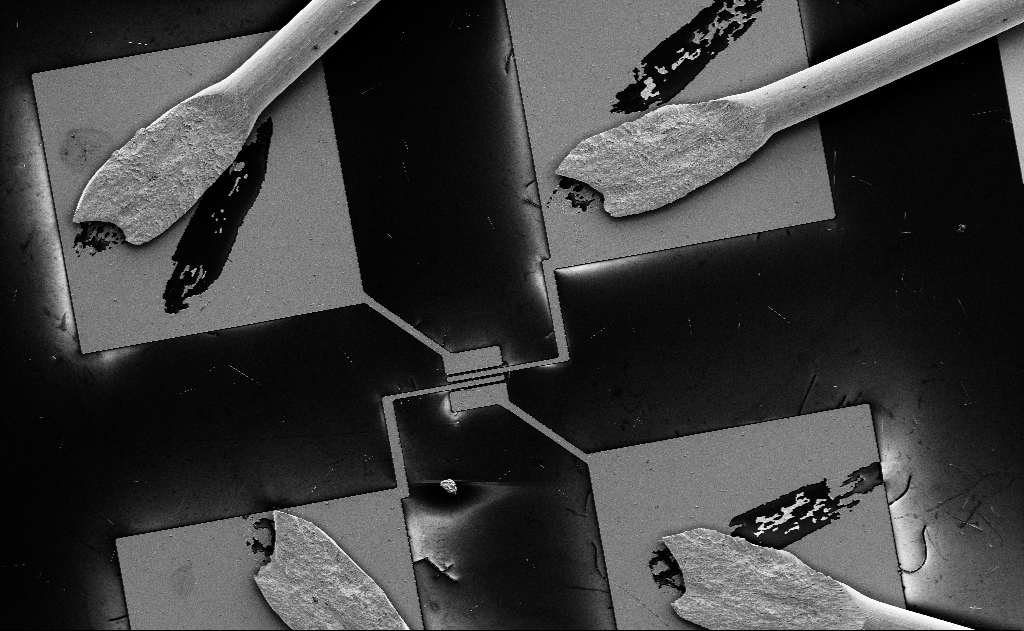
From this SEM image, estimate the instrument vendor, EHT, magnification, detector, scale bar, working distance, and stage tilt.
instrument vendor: Zeiss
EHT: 5 kV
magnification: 0.701 K X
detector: SE2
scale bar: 100000 nm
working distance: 18 mm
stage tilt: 0°